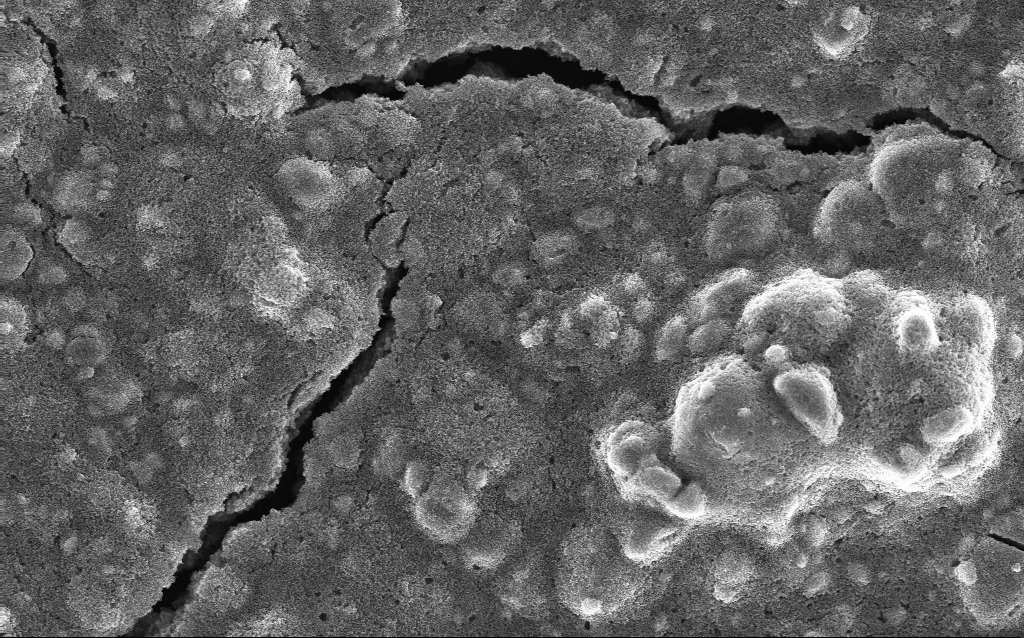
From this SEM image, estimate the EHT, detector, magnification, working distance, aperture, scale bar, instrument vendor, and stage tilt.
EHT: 10 kV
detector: InLens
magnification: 5 K X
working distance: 2.6 mm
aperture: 30 µm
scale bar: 10000 nm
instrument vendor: Zeiss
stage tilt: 0°